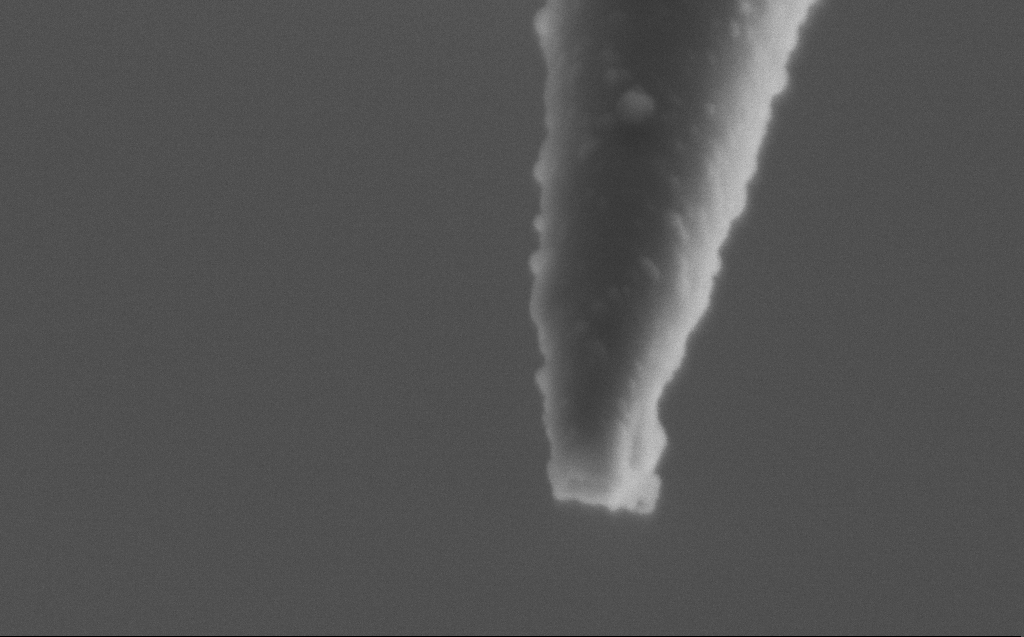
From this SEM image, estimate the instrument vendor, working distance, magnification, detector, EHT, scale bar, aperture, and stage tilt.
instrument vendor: Zeiss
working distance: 4 mm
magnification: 250 K X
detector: SE2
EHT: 2 kV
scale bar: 200 nm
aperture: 30 µm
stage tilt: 45°